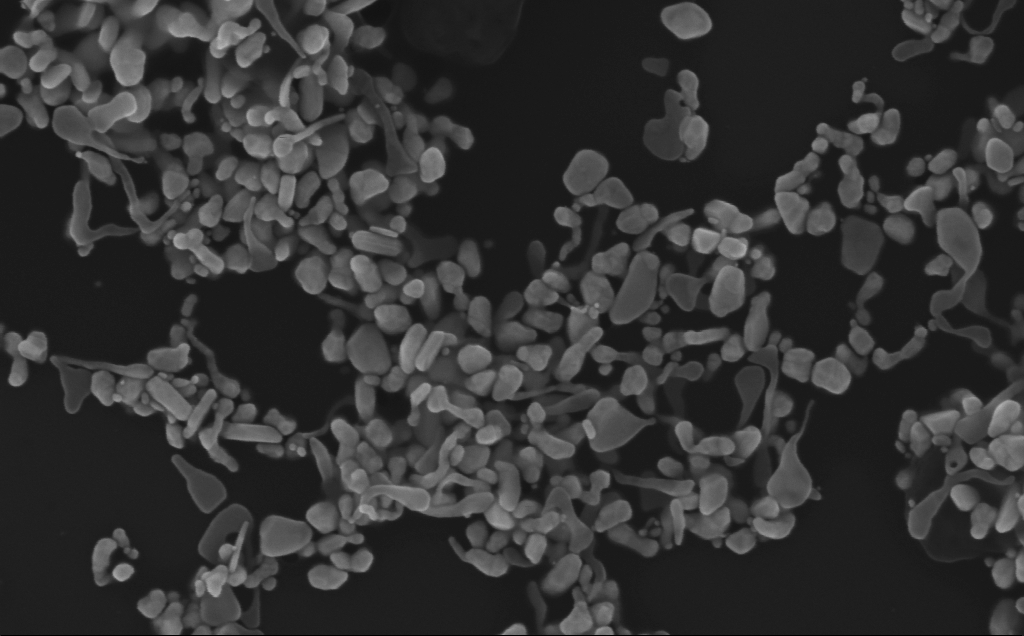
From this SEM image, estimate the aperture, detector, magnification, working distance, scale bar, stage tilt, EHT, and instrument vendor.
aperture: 30 µm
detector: InLens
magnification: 166.07 K X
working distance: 3 mm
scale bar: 200 nm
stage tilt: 0°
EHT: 10 kV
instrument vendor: Zeiss